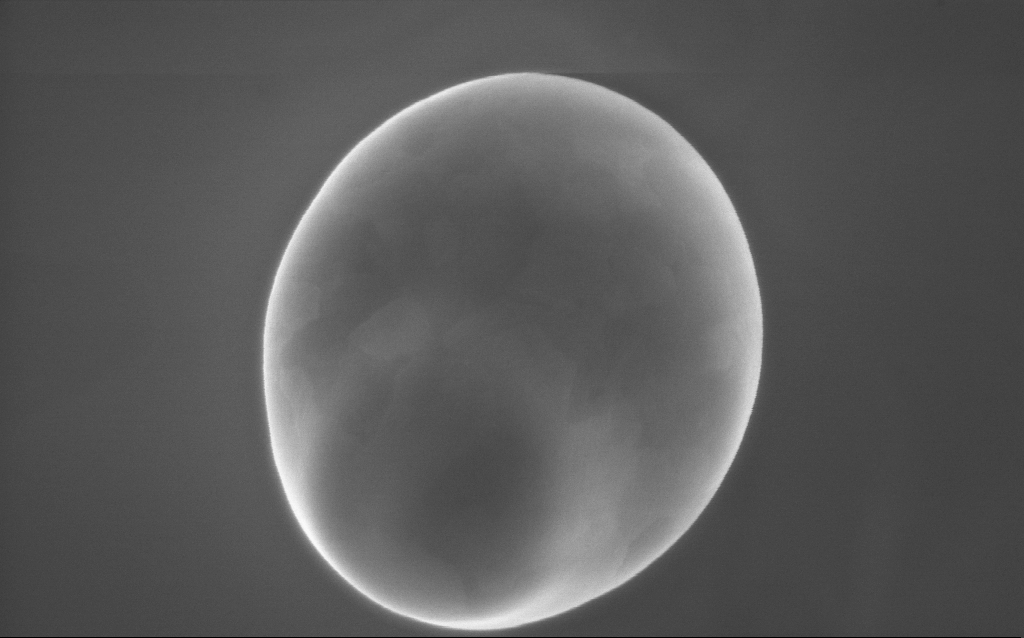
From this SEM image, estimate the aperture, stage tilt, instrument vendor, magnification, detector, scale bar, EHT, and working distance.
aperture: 30 µm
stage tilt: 0°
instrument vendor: Zeiss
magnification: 71 K X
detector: InLens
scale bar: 1000 nm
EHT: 10 kV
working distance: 3 mm